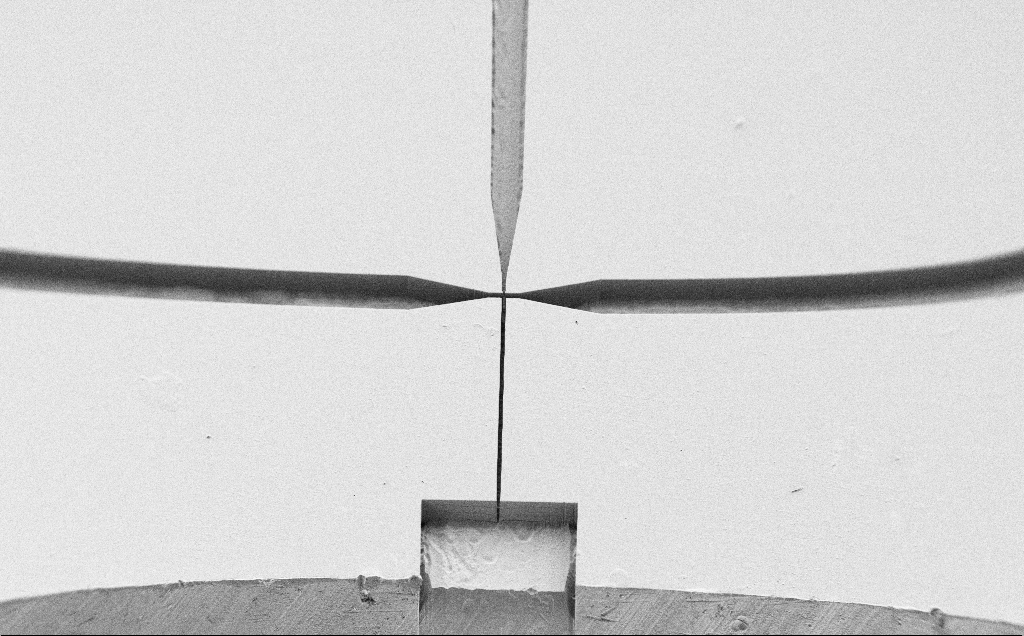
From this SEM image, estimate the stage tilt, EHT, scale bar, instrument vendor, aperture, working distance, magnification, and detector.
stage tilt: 45°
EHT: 1.2 kV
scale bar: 200000 nm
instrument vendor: Zeiss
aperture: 30 µm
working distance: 5 mm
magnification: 0.145 K X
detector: SE2